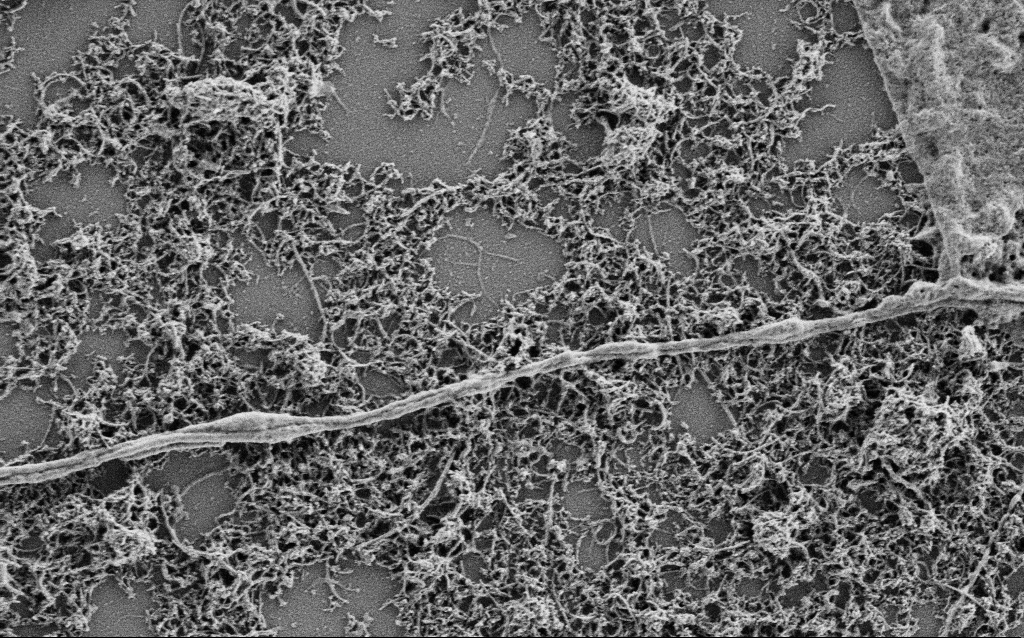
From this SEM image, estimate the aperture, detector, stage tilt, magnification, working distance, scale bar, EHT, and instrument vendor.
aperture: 30 µm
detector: SE2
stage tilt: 0°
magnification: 20 K X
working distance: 4 mm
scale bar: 1000 nm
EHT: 1 kV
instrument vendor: Zeiss